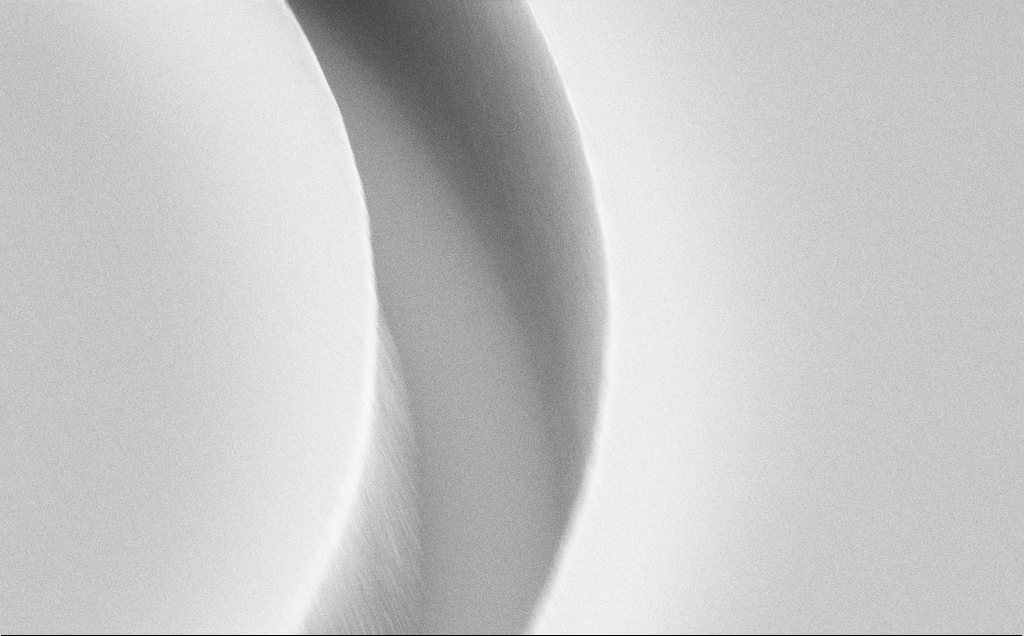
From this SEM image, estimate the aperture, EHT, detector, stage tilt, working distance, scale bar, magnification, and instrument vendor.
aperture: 30 µm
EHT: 10 kV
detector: SE2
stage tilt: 45°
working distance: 11 mm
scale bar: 1000 nm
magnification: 52.12 K X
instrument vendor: Zeiss